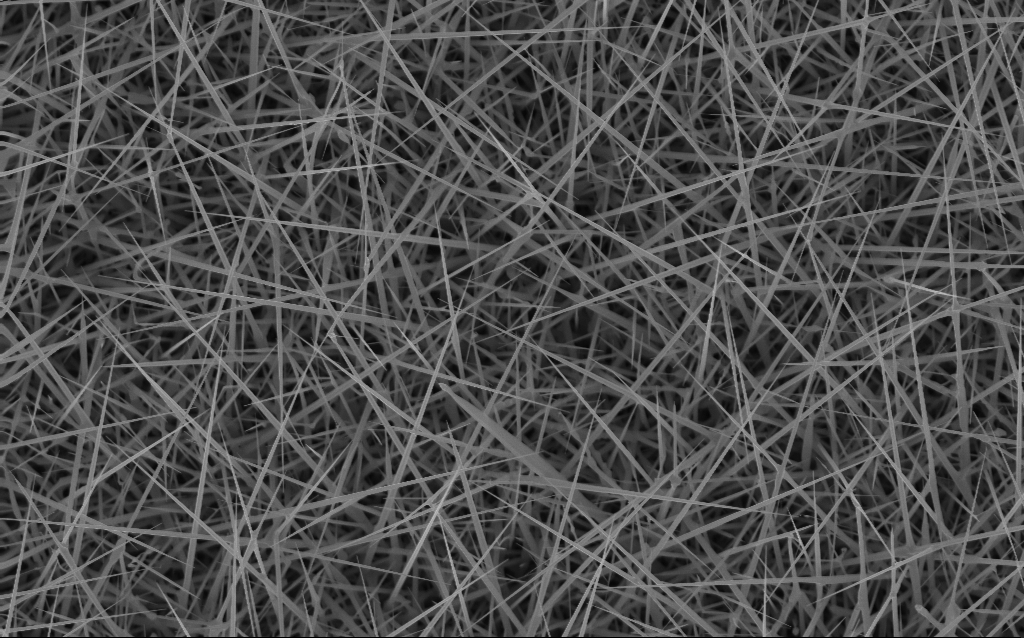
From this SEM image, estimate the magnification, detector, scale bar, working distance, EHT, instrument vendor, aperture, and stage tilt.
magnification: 10 K X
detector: InLens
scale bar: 2000 nm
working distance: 4 mm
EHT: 10 kV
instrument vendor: Zeiss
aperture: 30 µm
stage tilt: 0°